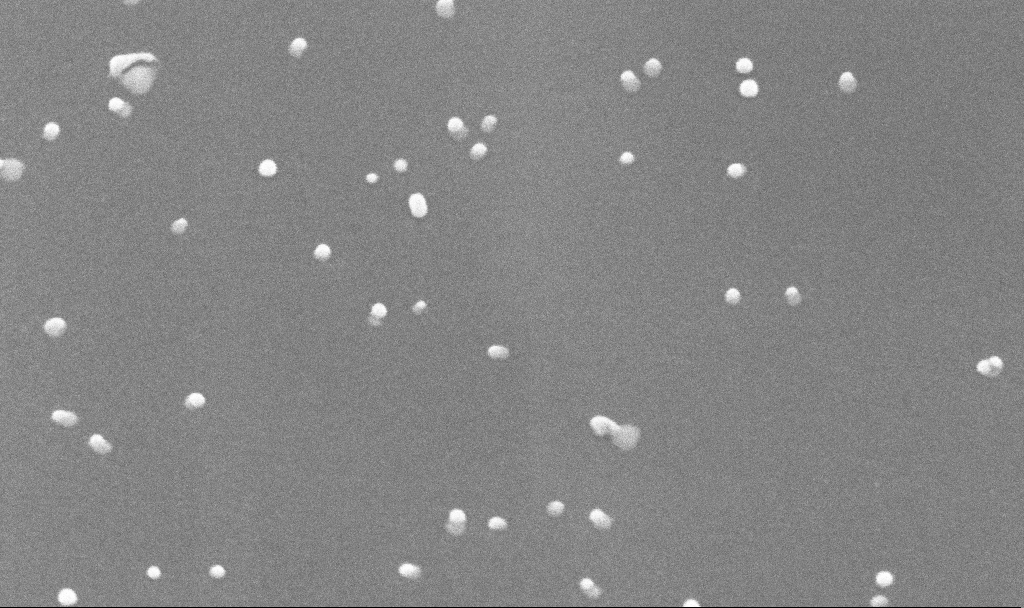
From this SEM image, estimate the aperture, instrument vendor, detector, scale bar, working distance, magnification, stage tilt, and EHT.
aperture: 30 µm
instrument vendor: Zeiss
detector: InLens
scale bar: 200 nm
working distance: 3.8 mm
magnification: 250 K X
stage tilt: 45°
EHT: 10 kV